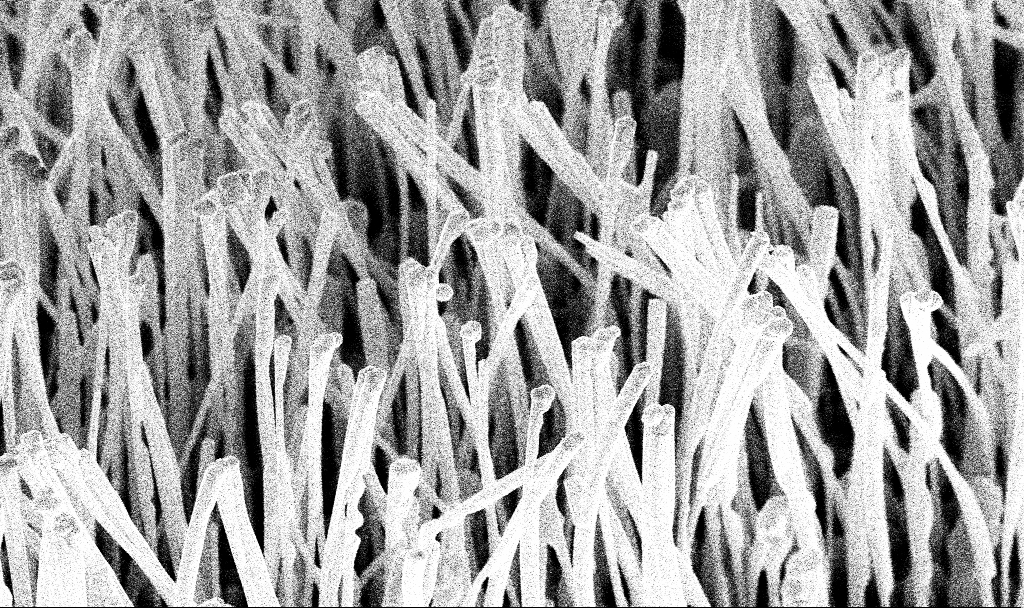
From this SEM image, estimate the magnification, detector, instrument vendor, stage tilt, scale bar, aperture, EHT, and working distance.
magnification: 31.1 K X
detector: InLens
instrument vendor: Zeiss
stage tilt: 45°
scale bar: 1000 nm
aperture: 30 µm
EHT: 10 kV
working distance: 7.2 mm